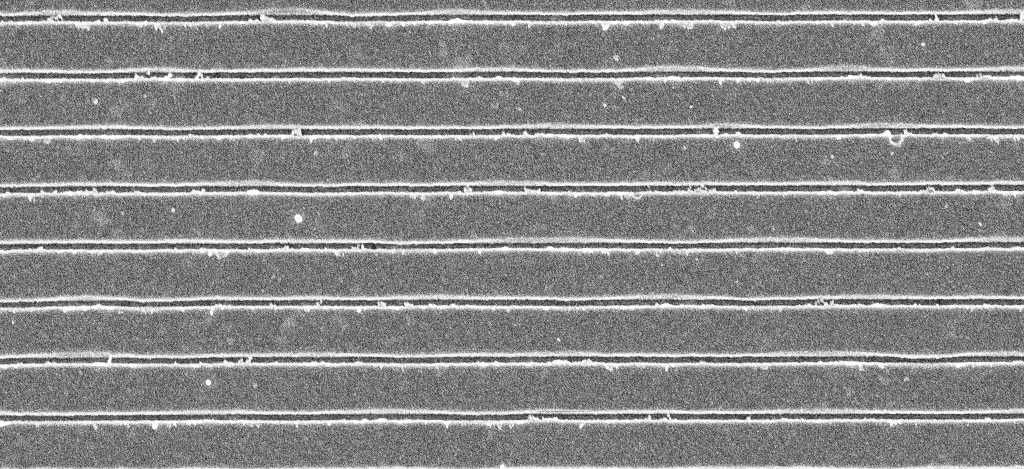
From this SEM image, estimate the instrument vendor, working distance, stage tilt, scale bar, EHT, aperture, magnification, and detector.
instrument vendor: Zeiss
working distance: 5.2 mm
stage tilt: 0°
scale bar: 2000 nm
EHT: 5 kV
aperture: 30 µm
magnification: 30.05 K X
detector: InLens